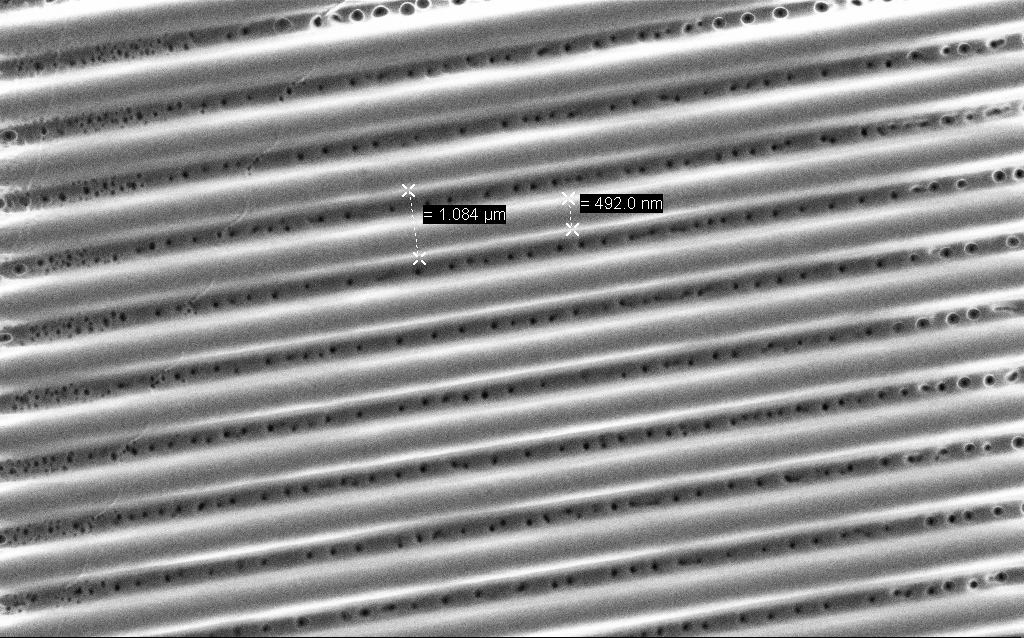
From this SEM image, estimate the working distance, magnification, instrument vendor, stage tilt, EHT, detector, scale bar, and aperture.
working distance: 6.4 mm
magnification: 23.33 K X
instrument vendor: Zeiss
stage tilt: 0°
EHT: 1.5 kV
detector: SE2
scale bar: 1000 nm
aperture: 30 µm